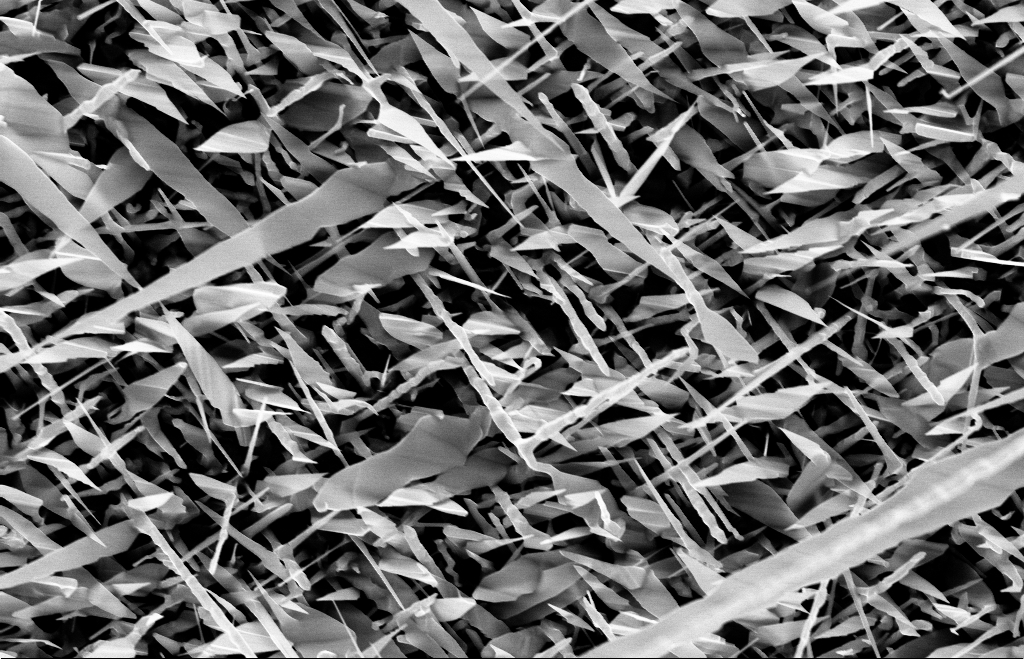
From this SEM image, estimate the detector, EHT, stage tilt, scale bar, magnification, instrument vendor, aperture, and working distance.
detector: InLens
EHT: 10 kV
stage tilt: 0°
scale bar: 1000 nm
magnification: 20 K X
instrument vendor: Zeiss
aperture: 30 µm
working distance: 8 mm